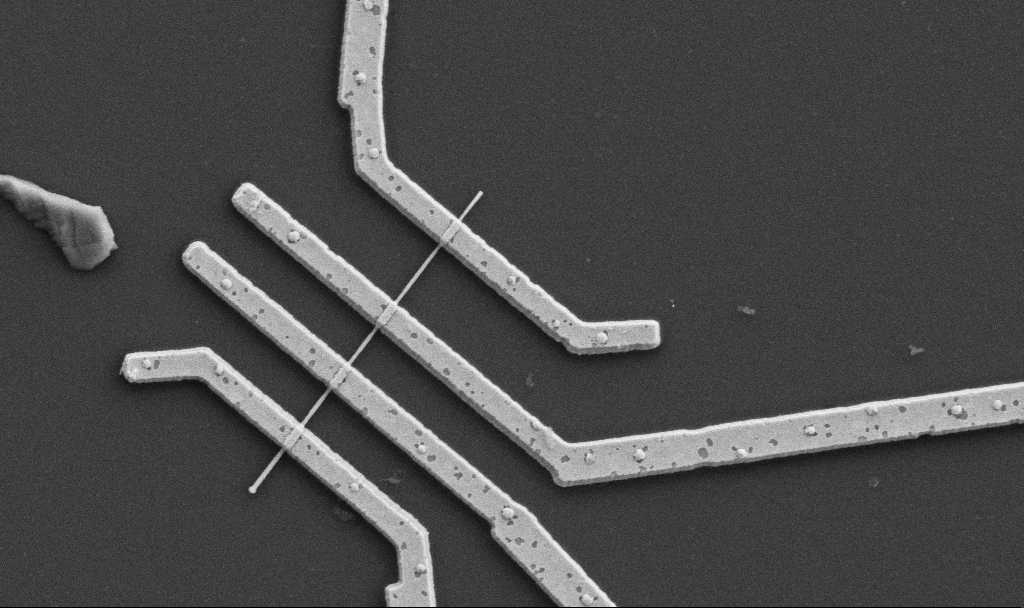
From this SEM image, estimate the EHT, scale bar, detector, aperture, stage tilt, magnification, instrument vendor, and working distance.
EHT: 5 kV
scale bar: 1000 nm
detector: SE2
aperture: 30 µm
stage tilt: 0°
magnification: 20 K X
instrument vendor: Zeiss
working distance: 10.6 mm